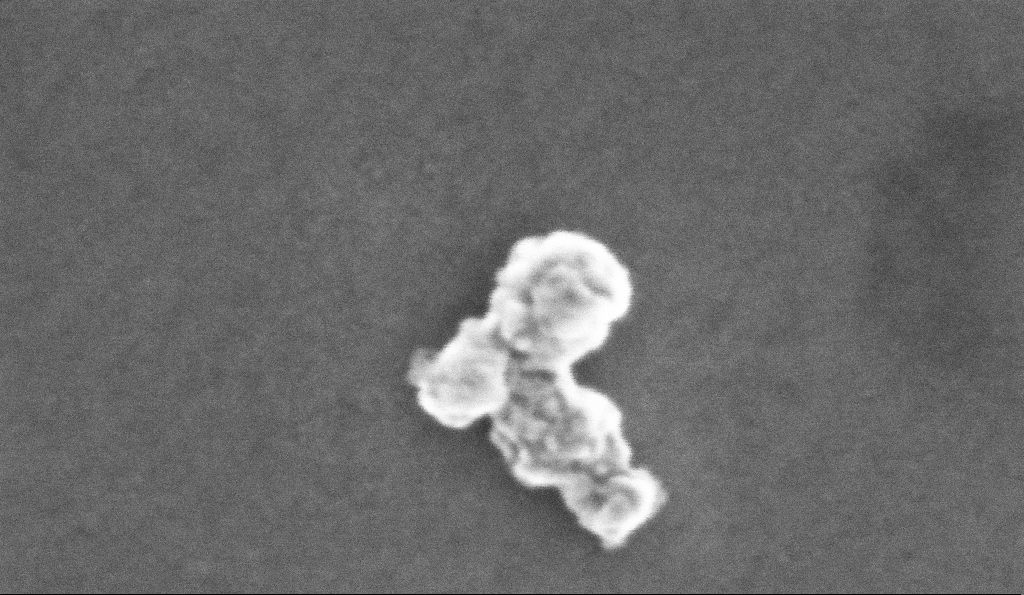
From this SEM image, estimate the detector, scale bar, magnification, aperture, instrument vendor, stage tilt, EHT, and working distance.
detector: InLens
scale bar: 20 nm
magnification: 775.13 K X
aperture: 30 µm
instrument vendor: Zeiss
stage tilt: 0°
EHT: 10 kV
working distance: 5.3 mm